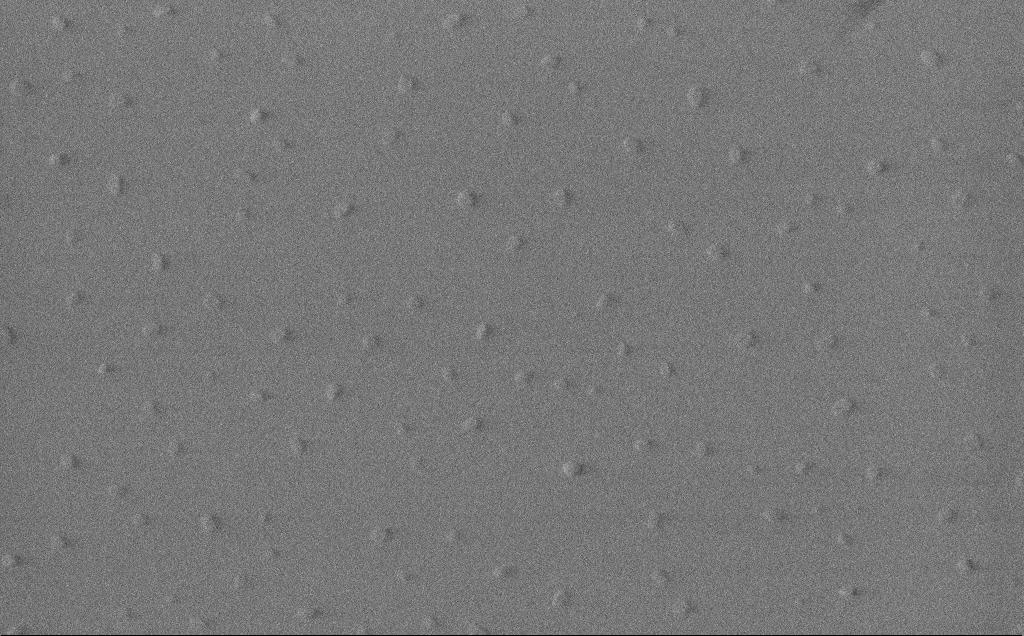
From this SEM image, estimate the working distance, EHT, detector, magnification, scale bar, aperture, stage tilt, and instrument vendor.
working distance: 3 mm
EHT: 1 kV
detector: InLens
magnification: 75.64 K X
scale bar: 200 nm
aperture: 30 µm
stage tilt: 0°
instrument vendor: Zeiss